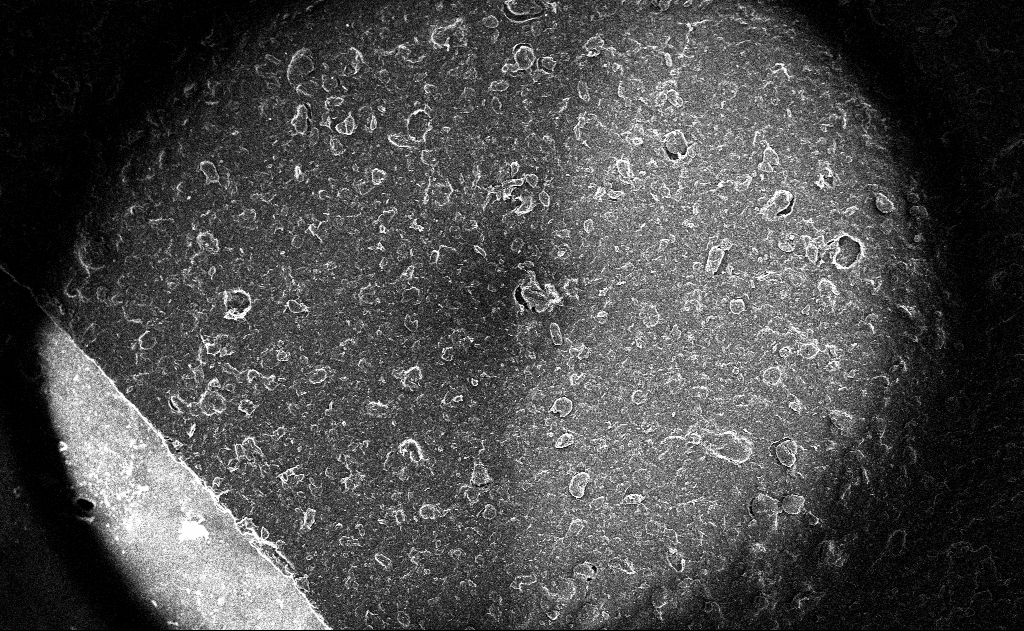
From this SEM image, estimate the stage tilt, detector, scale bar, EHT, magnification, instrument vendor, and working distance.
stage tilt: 0°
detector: InLens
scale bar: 200000 nm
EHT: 10 kV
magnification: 0.119 K X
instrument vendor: Zeiss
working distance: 3 mm